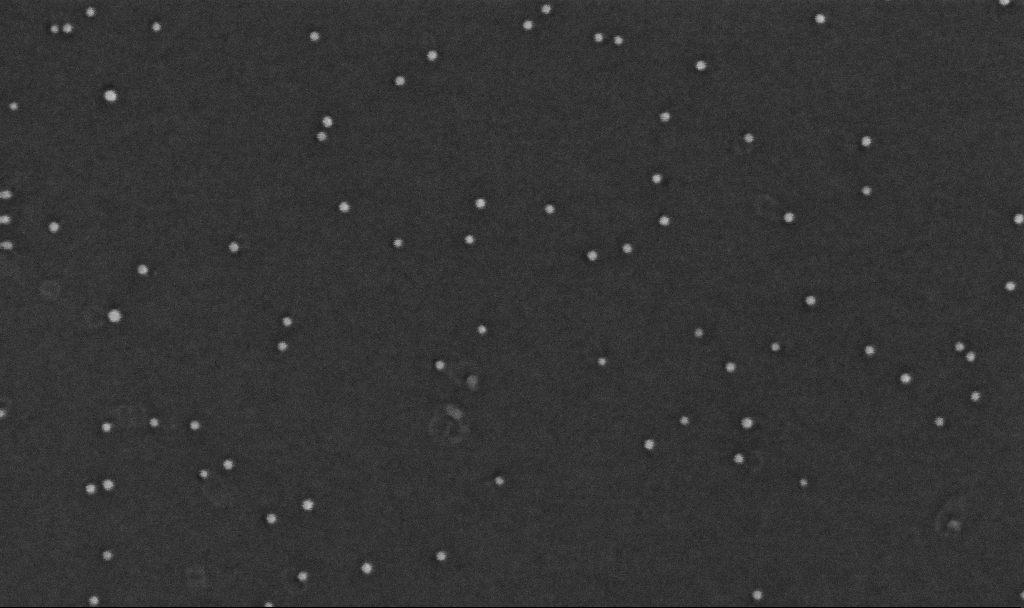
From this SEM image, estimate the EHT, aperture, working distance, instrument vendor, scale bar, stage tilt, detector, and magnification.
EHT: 10 kV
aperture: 30 µm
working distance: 3.3 mm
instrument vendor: Zeiss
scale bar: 100 nm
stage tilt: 0°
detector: InLens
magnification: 350 K X